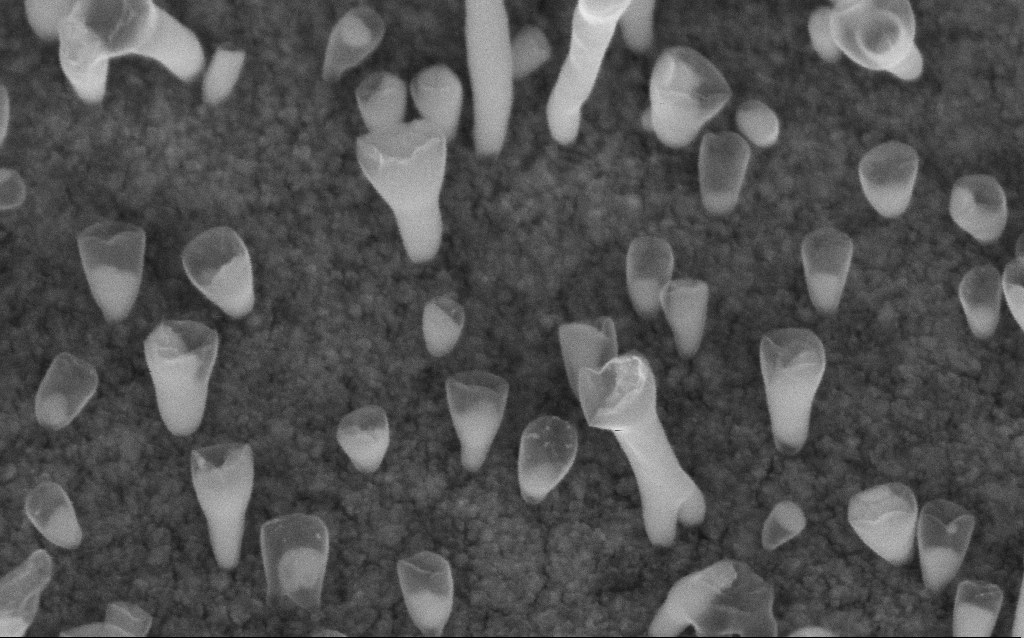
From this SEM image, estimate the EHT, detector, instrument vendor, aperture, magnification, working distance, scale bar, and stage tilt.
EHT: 5 kV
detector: InLens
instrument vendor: Zeiss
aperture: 30 µm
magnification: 200 K X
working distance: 6.7 mm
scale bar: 100 nm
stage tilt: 45°